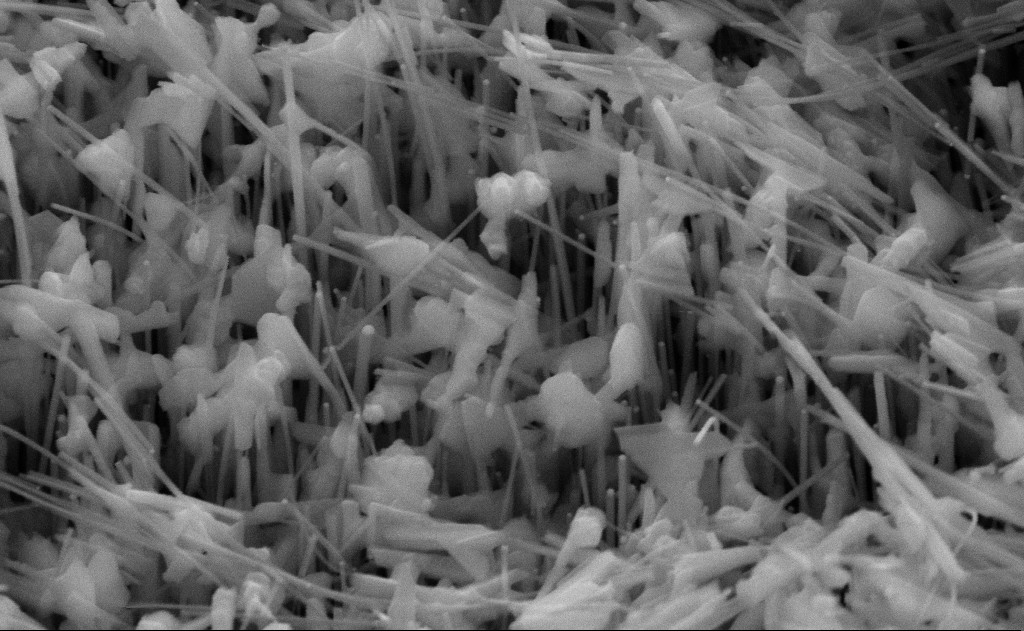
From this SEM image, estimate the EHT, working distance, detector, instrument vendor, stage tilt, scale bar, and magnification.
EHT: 10 kV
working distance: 11 mm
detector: SE2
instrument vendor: Zeiss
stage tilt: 45°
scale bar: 200 nm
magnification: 100 K X